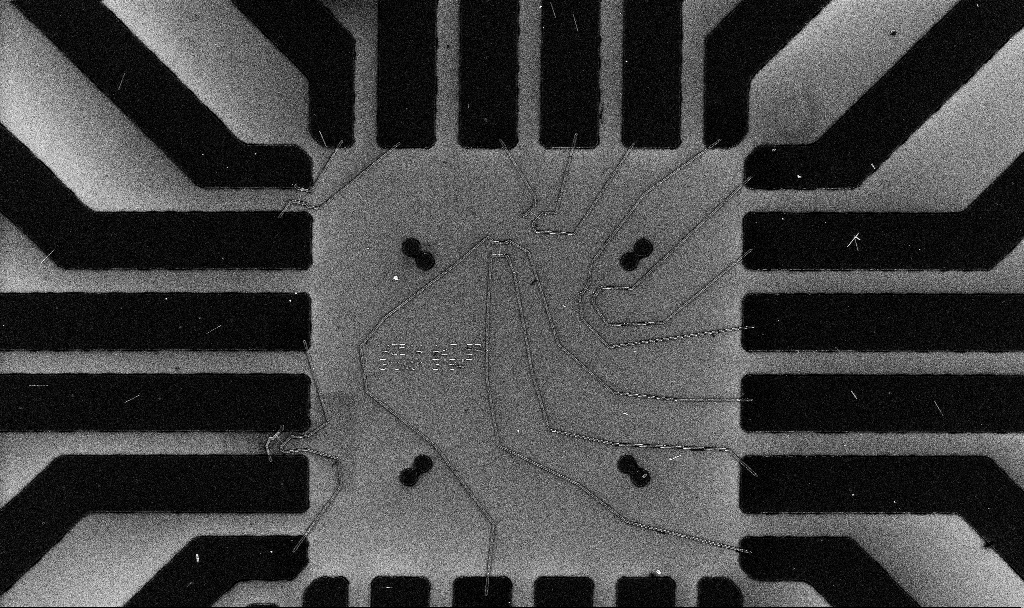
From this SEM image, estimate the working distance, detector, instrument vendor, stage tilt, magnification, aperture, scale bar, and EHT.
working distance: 6.7 mm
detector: InLens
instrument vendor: Zeiss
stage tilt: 0°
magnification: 1 K X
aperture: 30 µm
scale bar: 20000 nm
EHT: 10 kV